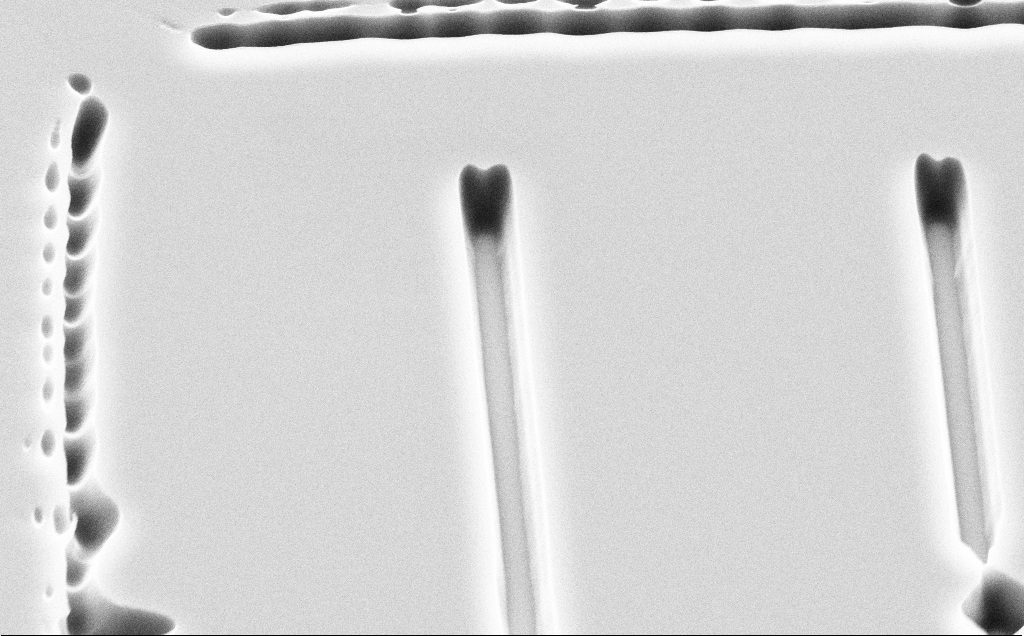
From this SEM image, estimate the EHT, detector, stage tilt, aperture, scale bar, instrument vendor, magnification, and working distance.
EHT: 10 kV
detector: SE2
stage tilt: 45°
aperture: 30 µm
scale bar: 2000 nm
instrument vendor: Zeiss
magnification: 8.31 K X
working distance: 13 mm